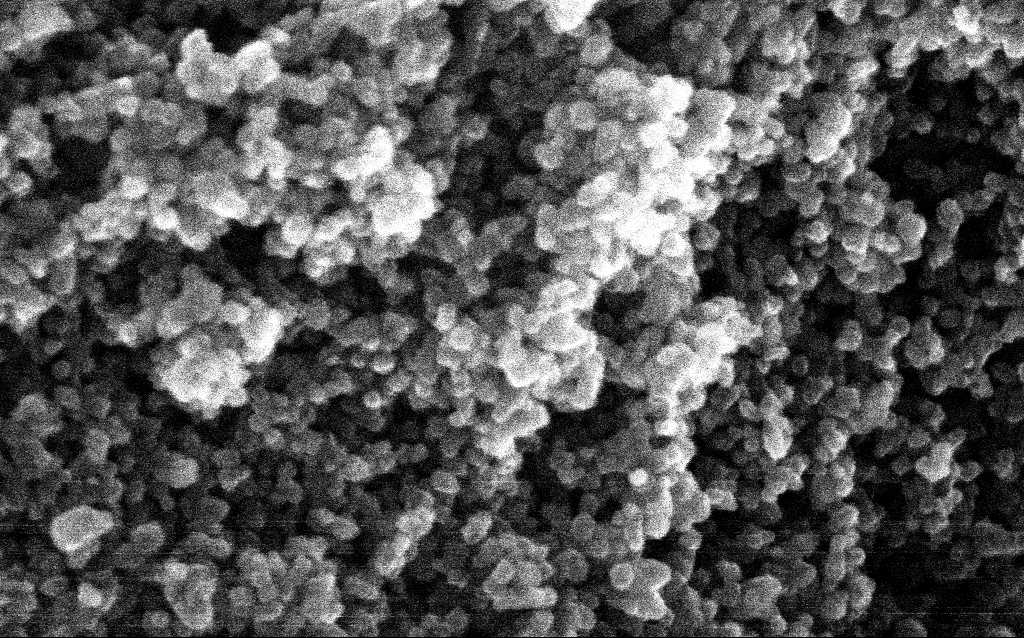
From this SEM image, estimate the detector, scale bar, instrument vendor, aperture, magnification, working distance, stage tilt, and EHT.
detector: InLens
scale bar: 100 nm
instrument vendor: Zeiss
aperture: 30 µm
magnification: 348.1 K X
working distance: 2.8 mm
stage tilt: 0°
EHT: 10 kV